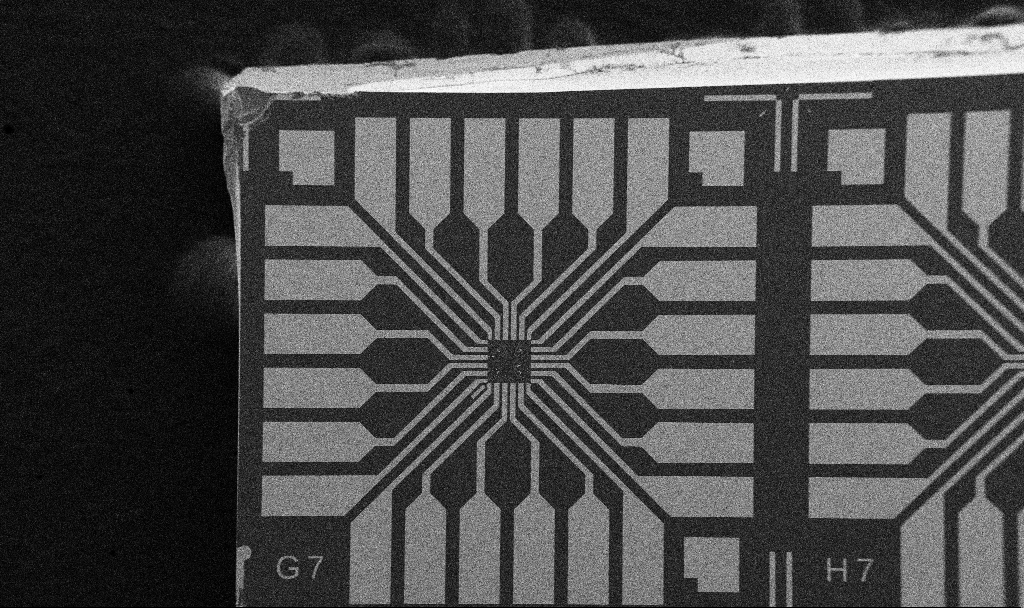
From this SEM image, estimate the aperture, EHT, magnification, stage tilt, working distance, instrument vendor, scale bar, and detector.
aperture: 30 µm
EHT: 5 kV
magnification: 0.1 K X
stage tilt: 0°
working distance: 10.7 mm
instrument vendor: Zeiss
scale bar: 200000 nm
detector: SE2